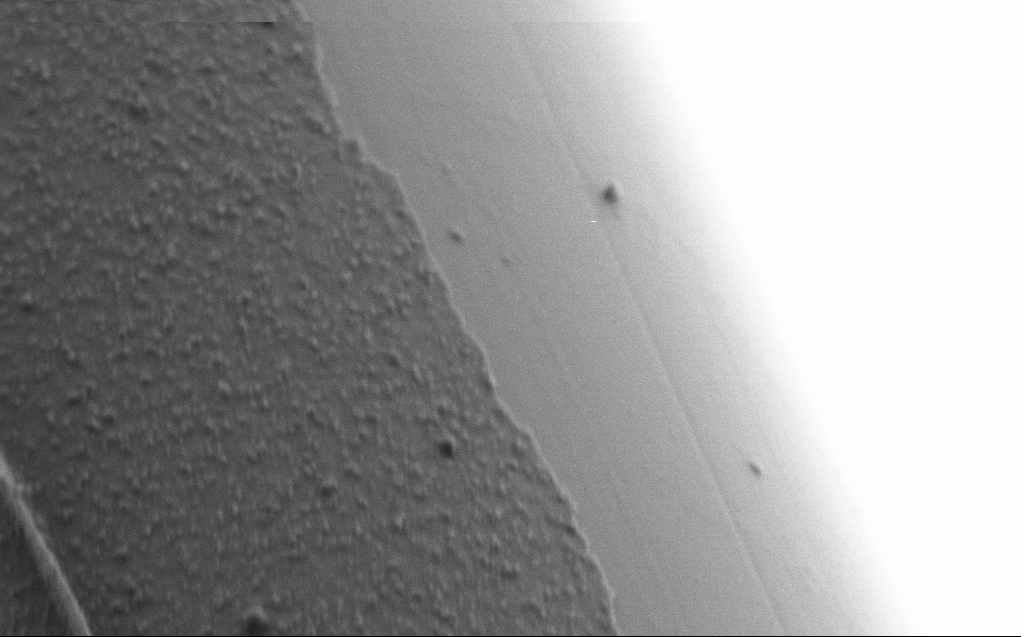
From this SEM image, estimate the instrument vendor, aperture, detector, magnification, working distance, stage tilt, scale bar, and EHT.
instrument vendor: Zeiss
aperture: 30 µm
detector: SE2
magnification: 75 K X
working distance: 4 mm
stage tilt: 45°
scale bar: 200 nm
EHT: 0.8 kV